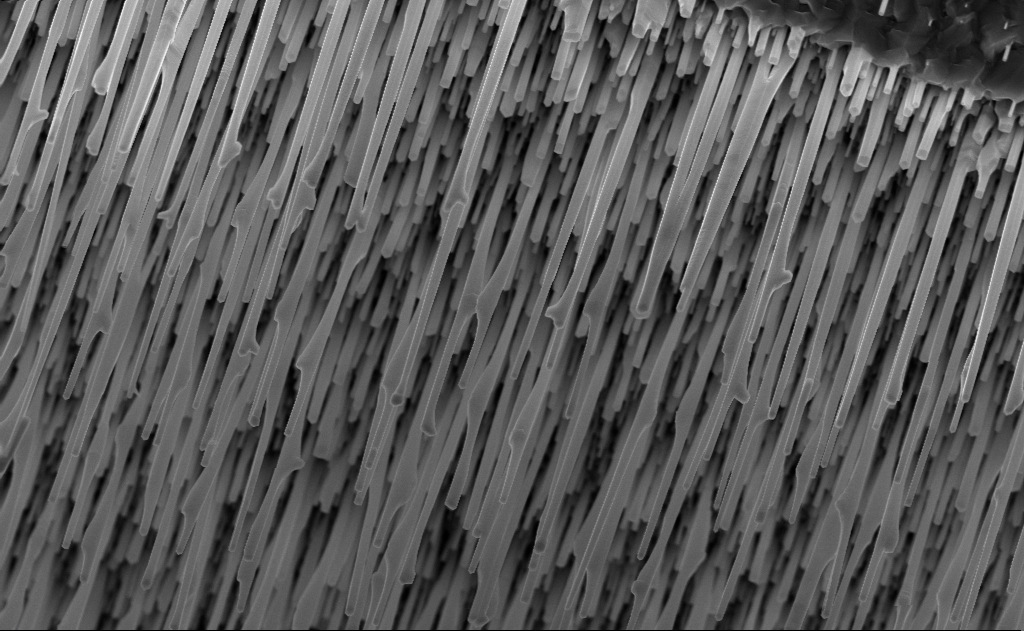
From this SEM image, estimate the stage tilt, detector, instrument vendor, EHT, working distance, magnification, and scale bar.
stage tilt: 0°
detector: InLens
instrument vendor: Zeiss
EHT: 10 kV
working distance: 9 mm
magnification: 20 K X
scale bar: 1000 nm